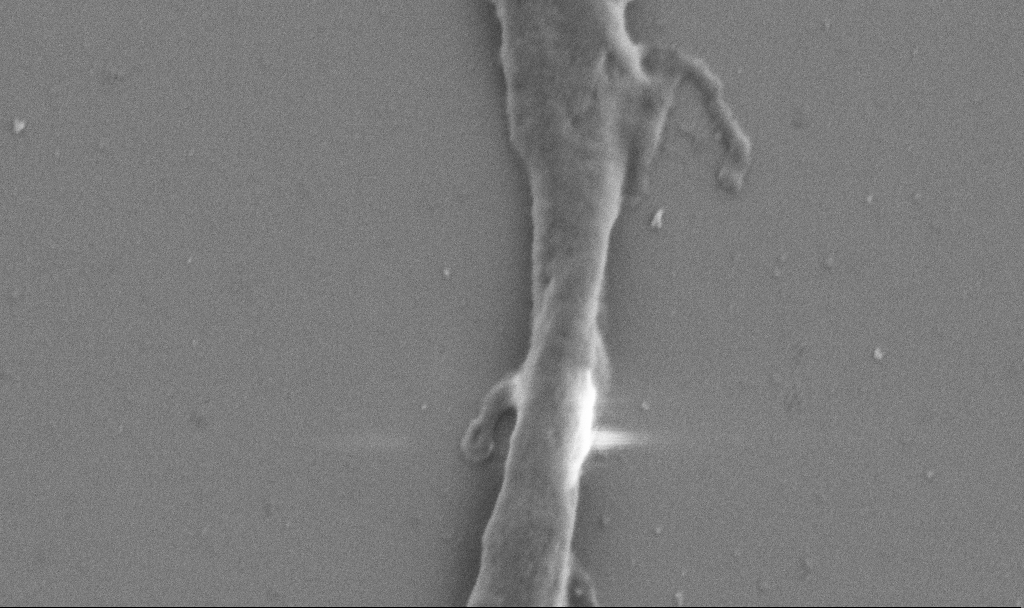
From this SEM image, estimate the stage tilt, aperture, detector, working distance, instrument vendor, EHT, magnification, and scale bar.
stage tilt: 0°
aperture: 30 µm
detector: SE2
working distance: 6.9 mm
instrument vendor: Zeiss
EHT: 1 kV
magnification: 50 K X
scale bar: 1000 nm